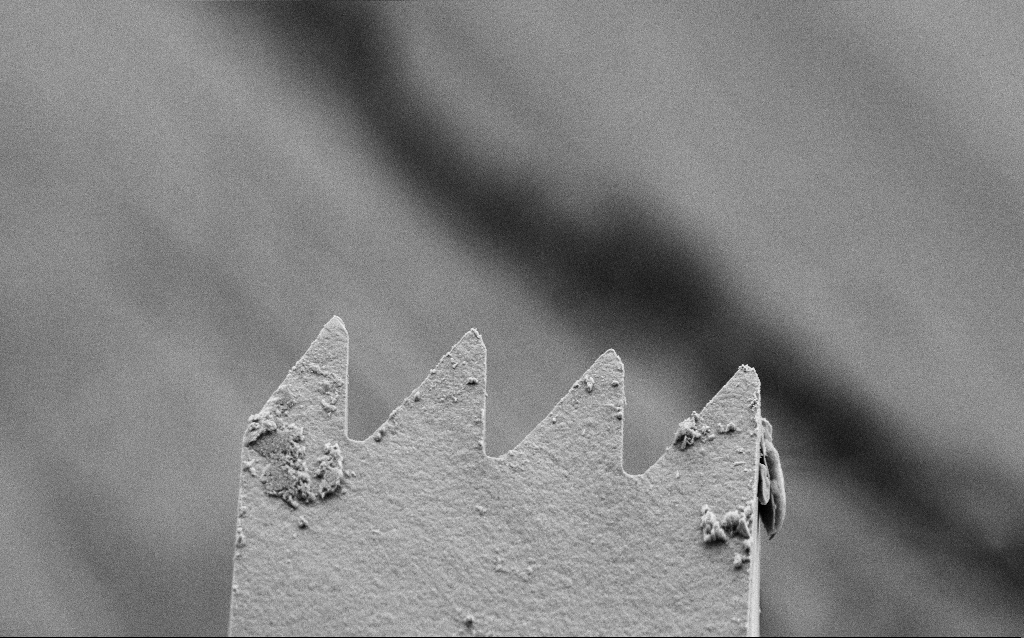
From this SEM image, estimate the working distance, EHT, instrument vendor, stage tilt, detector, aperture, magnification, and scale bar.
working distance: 4 mm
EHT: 5 kV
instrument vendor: Zeiss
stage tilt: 45°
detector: SE2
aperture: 30 µm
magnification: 4.58 K X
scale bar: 10000 nm